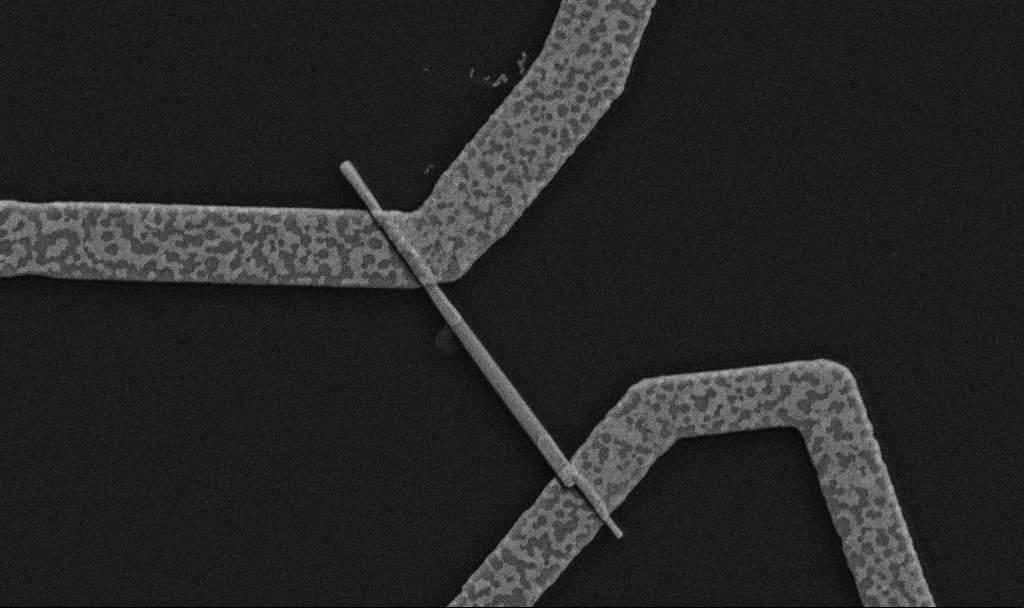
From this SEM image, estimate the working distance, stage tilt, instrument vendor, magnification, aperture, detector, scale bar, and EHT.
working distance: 10.7 mm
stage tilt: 0°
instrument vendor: Zeiss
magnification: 30 K X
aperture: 30 µm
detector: SE2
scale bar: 1000 nm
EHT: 5 kV